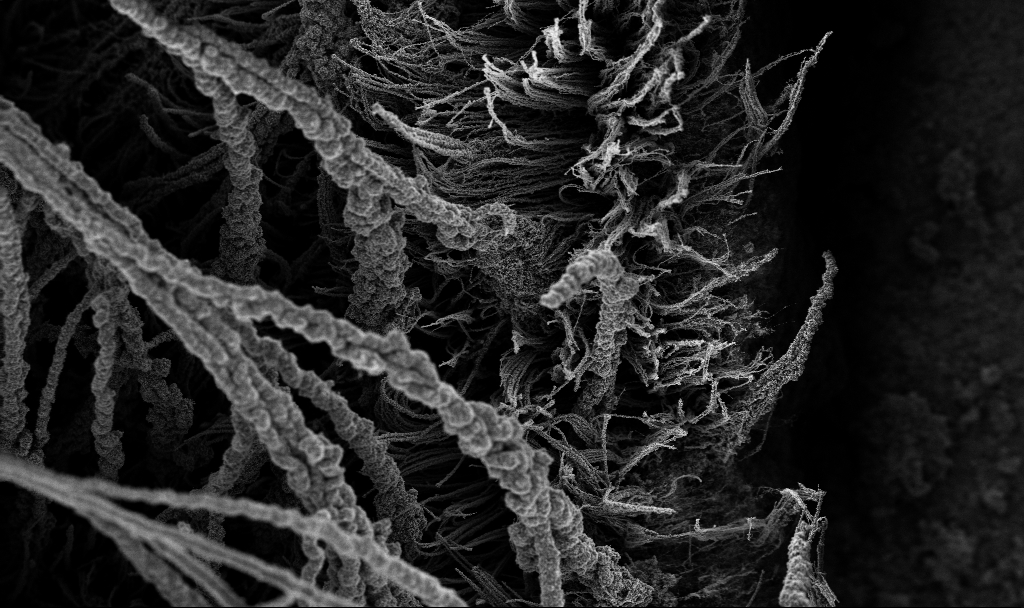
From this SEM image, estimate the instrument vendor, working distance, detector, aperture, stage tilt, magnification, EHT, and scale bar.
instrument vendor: Zeiss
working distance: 3.4 mm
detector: InLens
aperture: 30 µm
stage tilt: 0°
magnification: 0.25 K X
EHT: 3 kV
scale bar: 100000 nm